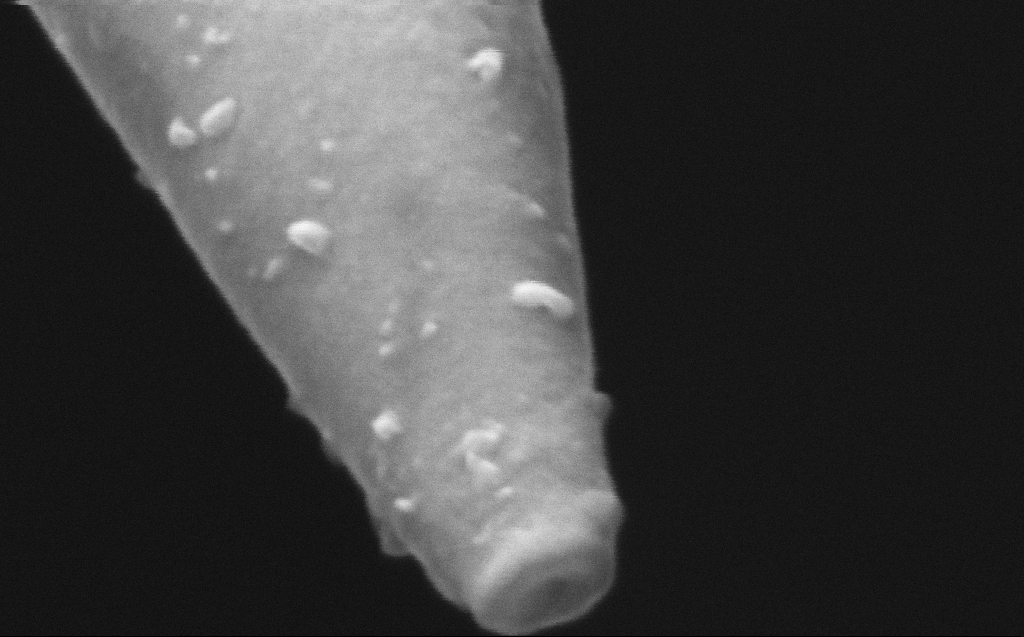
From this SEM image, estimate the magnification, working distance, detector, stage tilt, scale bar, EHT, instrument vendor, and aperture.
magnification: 500 K X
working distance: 6 mm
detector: InLens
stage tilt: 45°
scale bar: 100 nm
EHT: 5 kV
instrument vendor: Zeiss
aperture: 30 µm